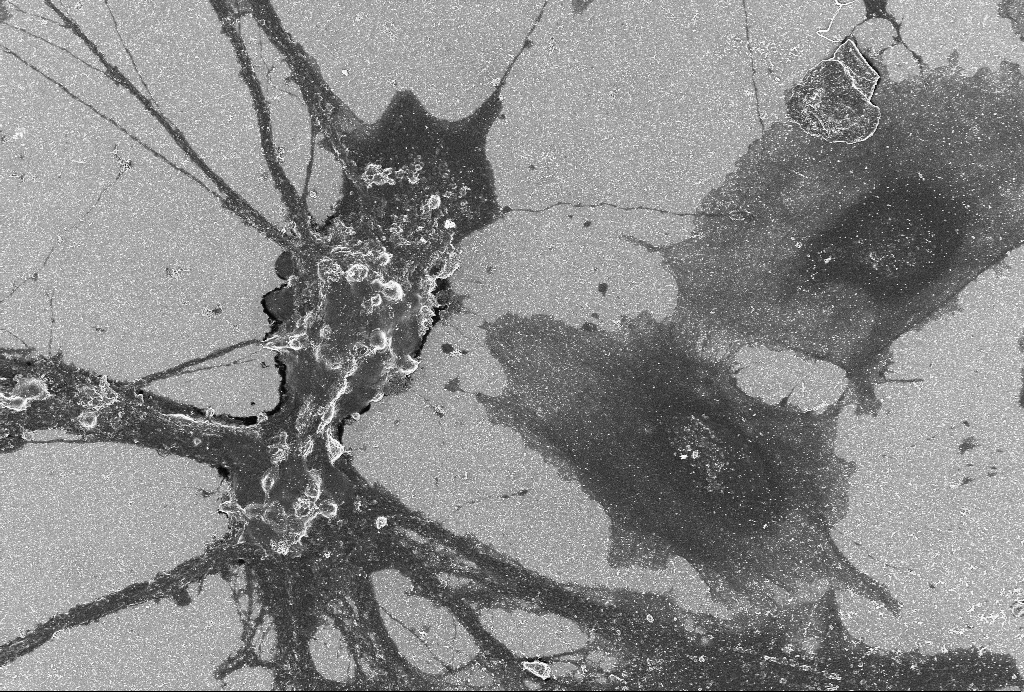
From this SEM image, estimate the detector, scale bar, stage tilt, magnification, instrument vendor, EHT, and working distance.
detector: SE2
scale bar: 20000 nm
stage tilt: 0°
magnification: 1 K X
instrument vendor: Zeiss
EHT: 4 kV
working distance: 6 mm